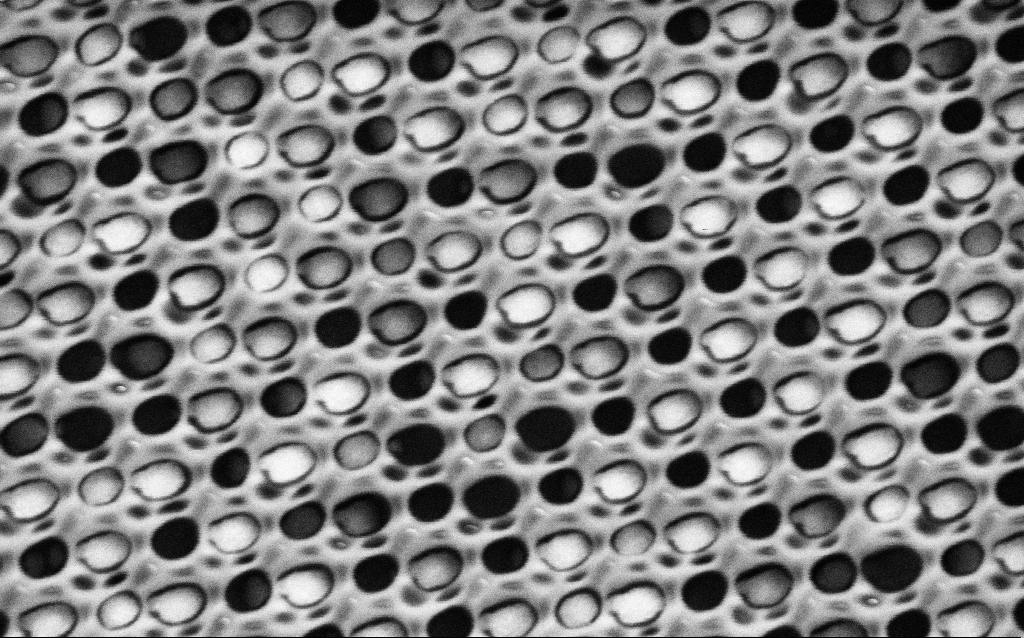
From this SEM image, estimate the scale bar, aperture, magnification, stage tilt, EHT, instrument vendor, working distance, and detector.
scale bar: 1000 nm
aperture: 30 µm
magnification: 72.21 K X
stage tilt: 0°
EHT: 1.5 kV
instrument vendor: Zeiss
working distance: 8.1 mm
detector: SE2